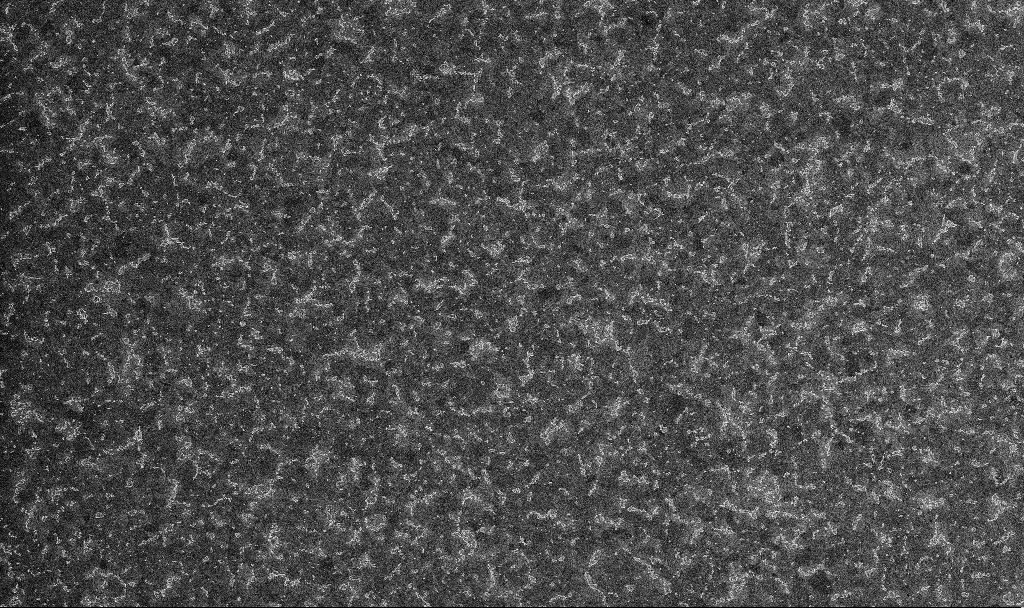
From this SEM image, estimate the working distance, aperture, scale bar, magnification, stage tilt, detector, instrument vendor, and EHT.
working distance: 3.3 mm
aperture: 30 µm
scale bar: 1000 nm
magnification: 20 K X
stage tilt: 0°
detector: InLens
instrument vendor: Zeiss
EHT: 10 kV